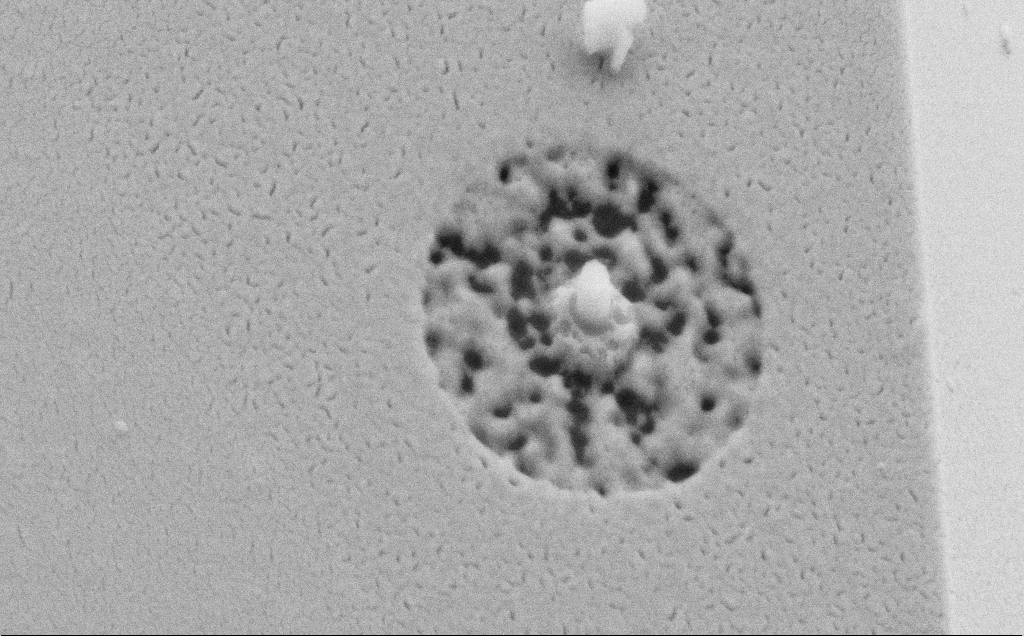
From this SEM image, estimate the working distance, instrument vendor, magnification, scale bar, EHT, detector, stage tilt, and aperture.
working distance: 3 mm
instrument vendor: Zeiss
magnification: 41.93 K X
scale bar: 1000 nm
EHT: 10 kV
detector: SE2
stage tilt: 44.2°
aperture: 30 µm